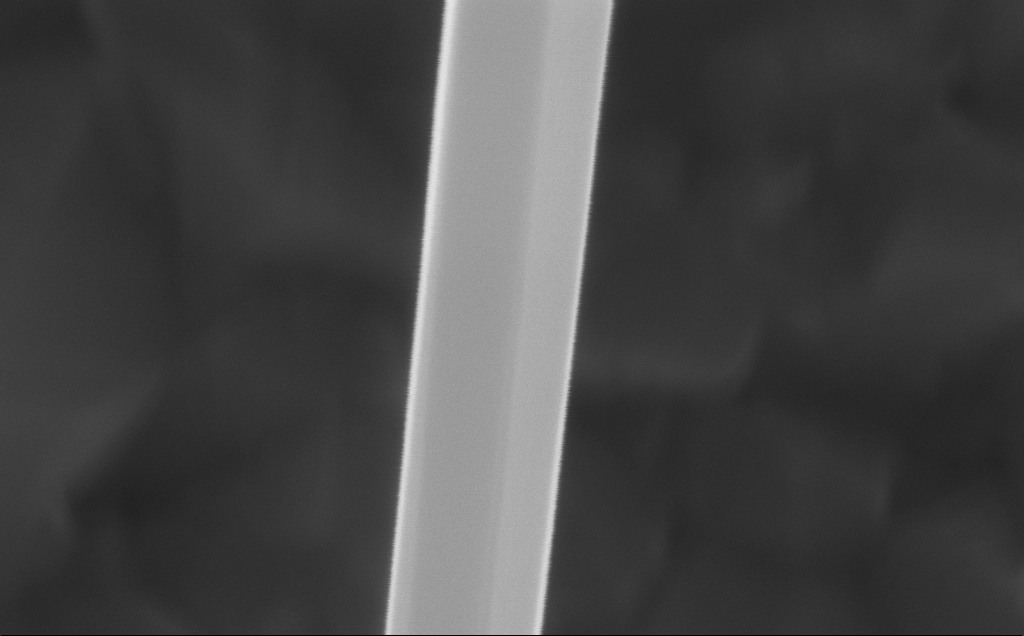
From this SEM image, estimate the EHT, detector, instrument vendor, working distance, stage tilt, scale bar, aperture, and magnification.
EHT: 10 kV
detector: InLens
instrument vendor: Zeiss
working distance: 5 mm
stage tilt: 0°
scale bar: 100 nm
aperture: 30 µm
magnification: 313.41 K X